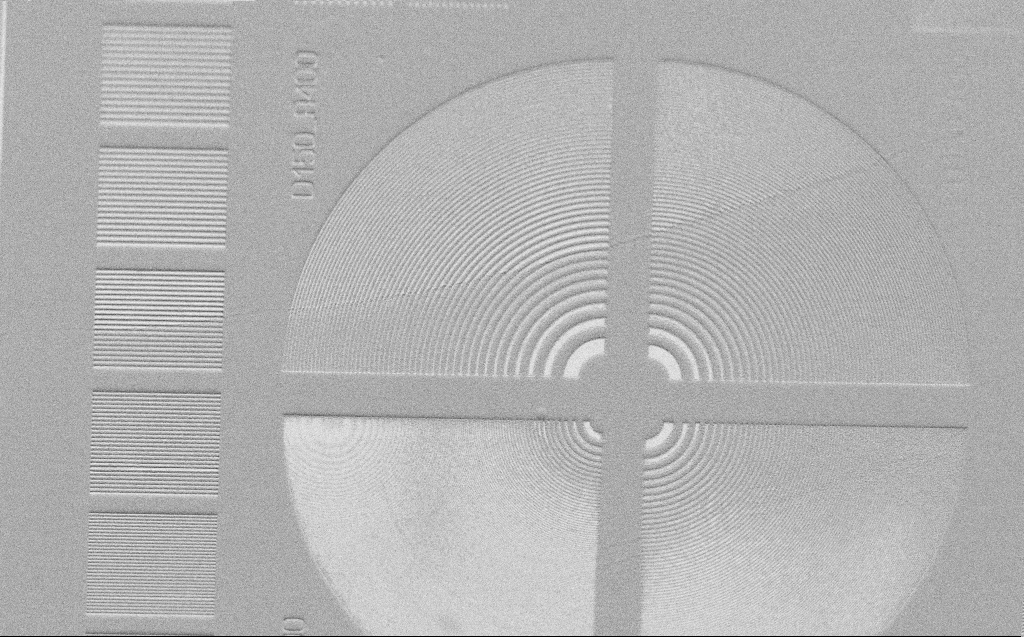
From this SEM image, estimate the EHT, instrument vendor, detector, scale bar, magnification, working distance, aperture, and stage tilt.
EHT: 1 kV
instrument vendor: Zeiss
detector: SE2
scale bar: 10000 nm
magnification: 1.59 K X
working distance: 5 mm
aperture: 30 µm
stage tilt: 45°